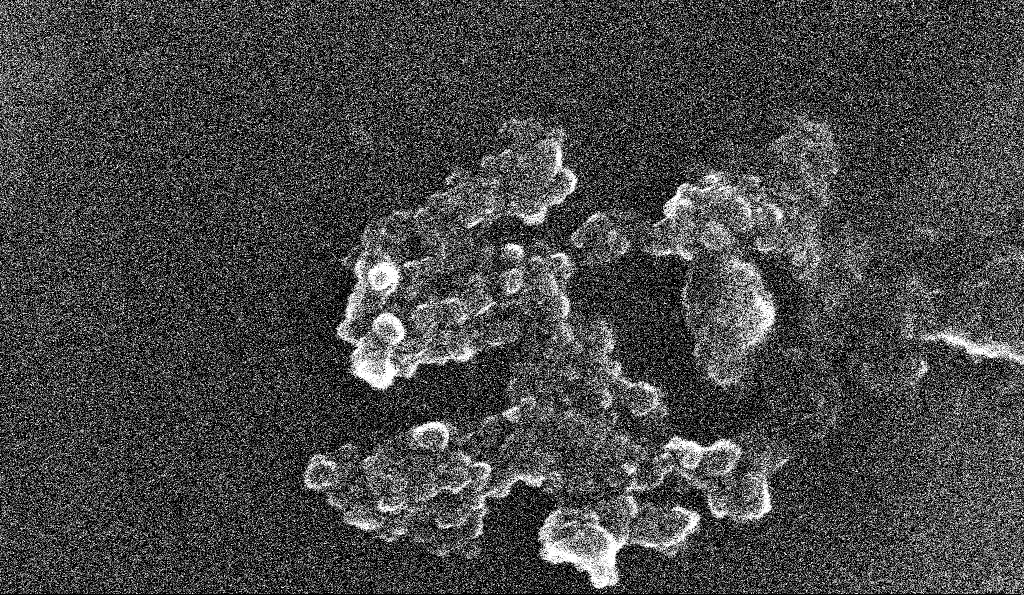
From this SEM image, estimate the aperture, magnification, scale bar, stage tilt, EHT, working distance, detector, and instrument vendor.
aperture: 30 µm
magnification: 172.24 K X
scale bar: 200 nm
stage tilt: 0°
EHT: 10 kV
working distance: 5.3 mm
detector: InLens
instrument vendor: Zeiss